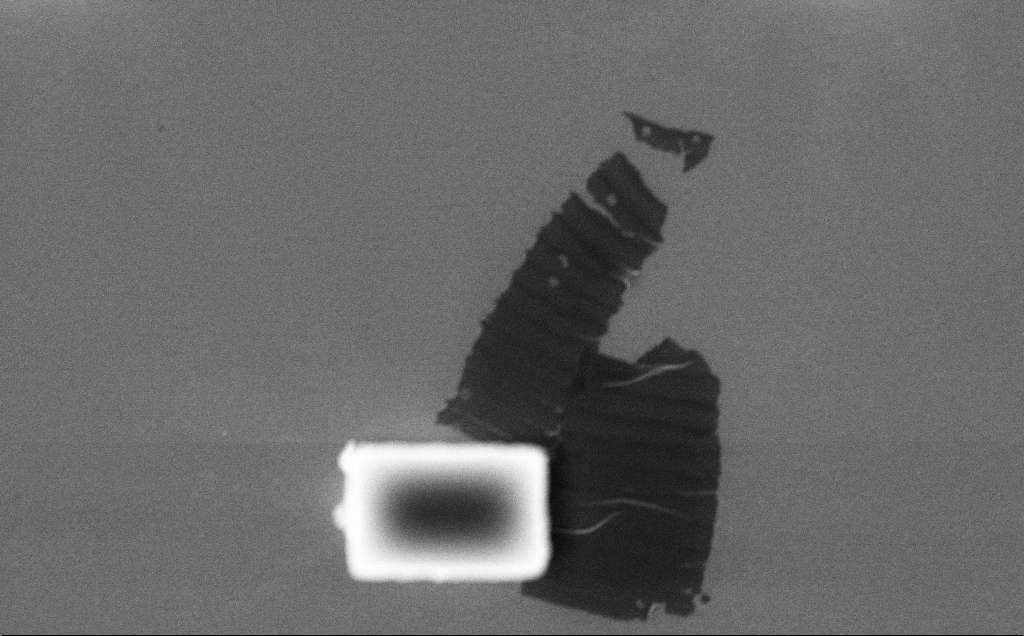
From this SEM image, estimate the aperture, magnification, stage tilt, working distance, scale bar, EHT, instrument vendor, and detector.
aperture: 30 µm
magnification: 23.92 K X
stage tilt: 0°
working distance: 3.2 mm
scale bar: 1000 nm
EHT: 10 kV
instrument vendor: Zeiss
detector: InLens